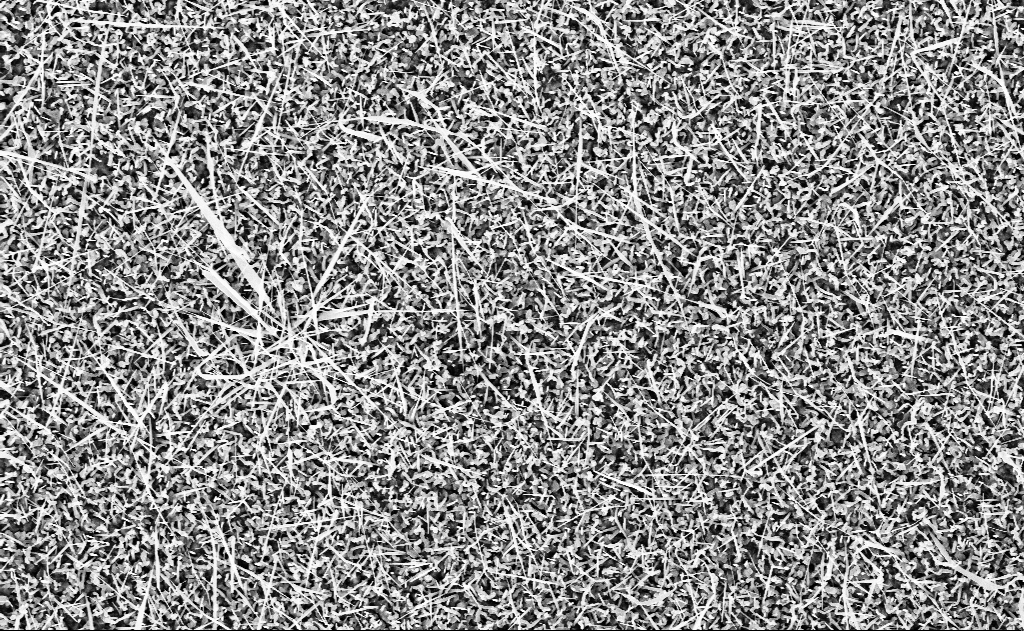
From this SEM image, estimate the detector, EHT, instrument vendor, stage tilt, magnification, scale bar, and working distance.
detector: InLens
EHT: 10 kV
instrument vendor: Zeiss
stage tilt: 0°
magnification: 10 K X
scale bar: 2000 nm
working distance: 15 mm